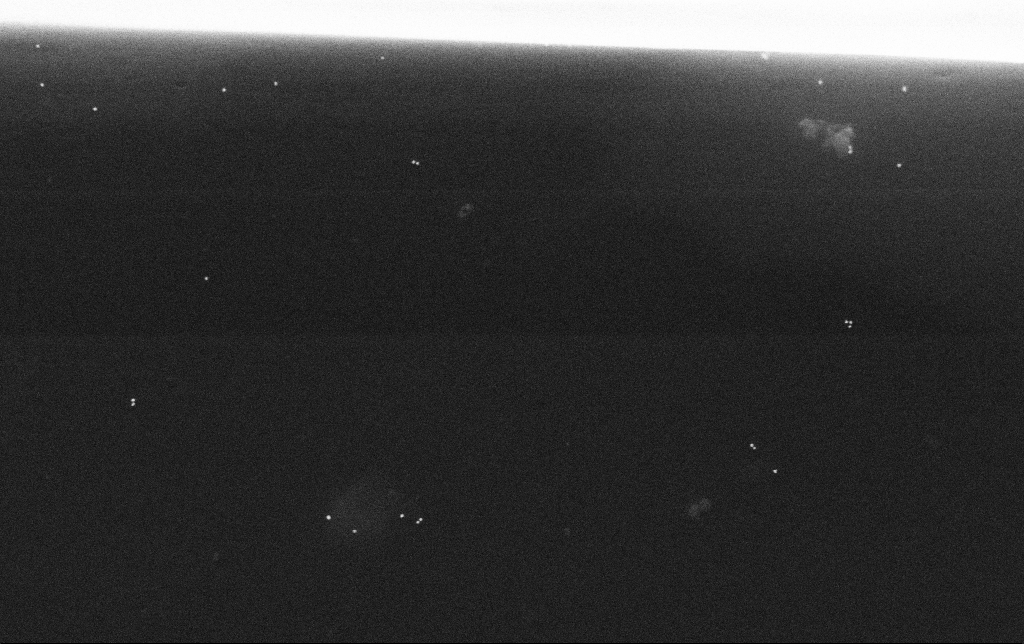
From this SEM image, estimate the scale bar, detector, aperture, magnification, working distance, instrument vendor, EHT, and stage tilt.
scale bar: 200 nm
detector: SE2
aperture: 30 µm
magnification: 100 K X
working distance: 10.8 mm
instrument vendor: Zeiss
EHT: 30 kV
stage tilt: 0°